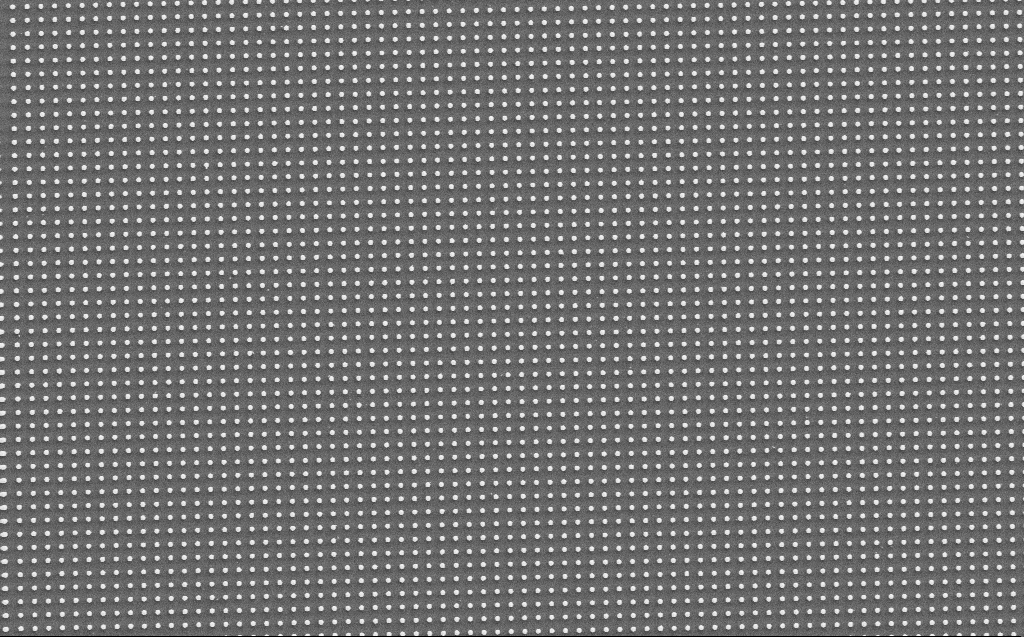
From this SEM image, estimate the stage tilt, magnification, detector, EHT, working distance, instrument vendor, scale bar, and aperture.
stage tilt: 0°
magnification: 4.78 K X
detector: SE2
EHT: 5 kV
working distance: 5 mm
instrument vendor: Zeiss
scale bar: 10000 nm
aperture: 30 µm